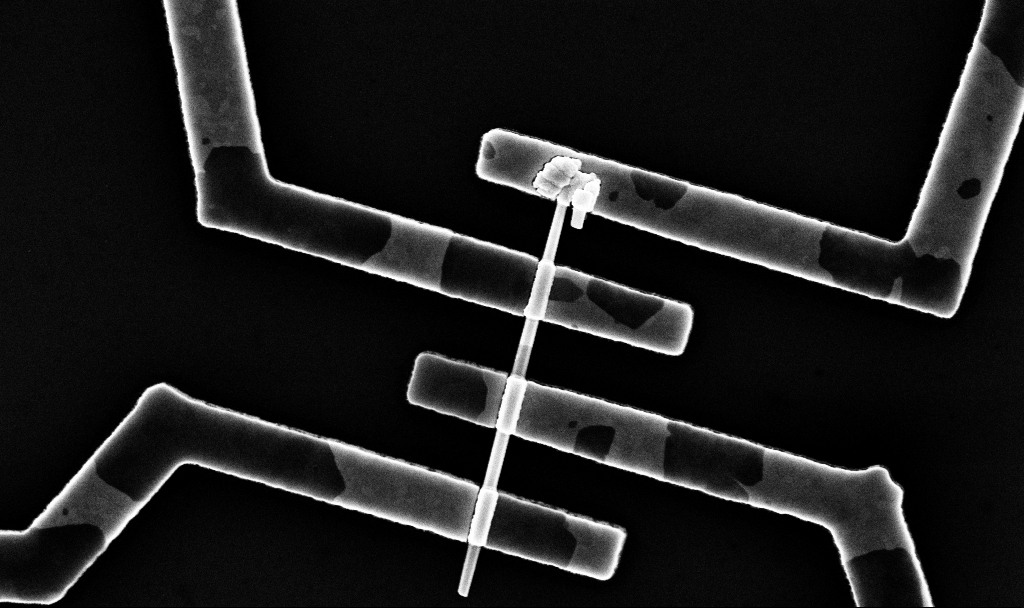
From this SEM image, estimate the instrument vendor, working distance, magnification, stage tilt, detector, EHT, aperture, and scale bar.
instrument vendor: Zeiss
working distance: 6.7 mm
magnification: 36.38 K X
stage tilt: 0°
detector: InLens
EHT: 10 kV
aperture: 30 µm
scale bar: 1000 nm